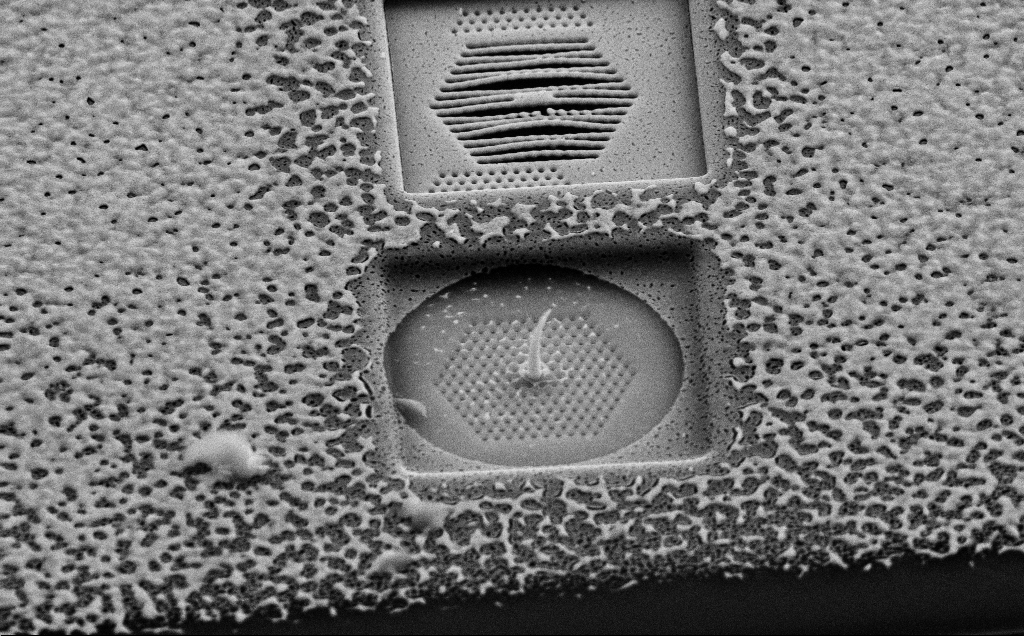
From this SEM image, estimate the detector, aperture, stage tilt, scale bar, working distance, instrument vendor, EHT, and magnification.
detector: SE2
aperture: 30 µm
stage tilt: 45°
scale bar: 2000 nm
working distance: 8 mm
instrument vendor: Zeiss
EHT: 10 kV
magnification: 18.22 K X